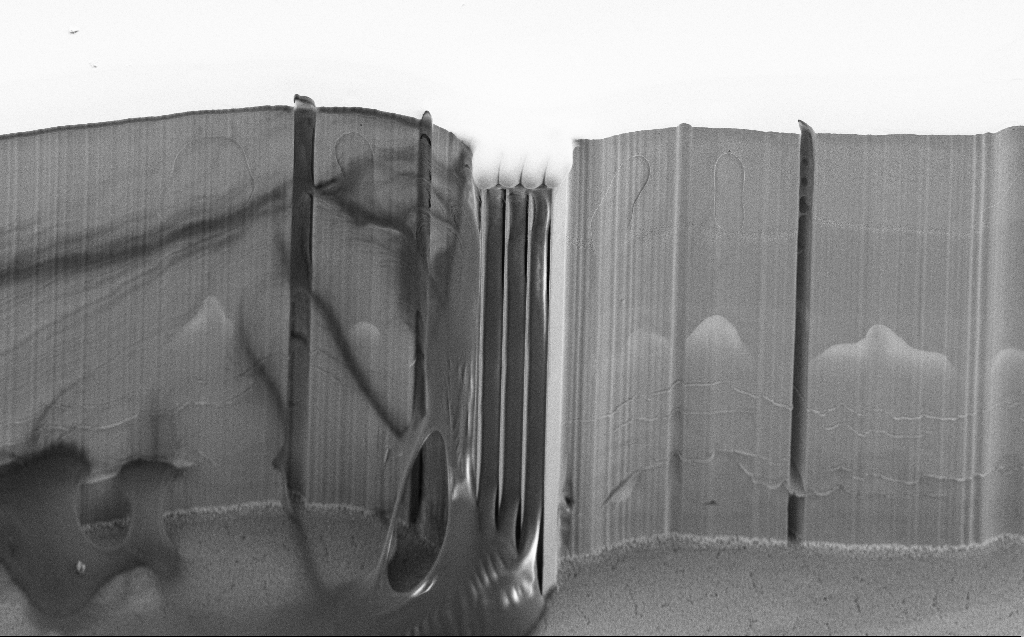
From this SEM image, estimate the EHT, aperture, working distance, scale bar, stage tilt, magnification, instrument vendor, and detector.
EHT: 10 kV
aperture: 30 µm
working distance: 6 mm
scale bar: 20000 nm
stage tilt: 45°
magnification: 0.737 K X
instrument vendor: Zeiss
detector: SE2